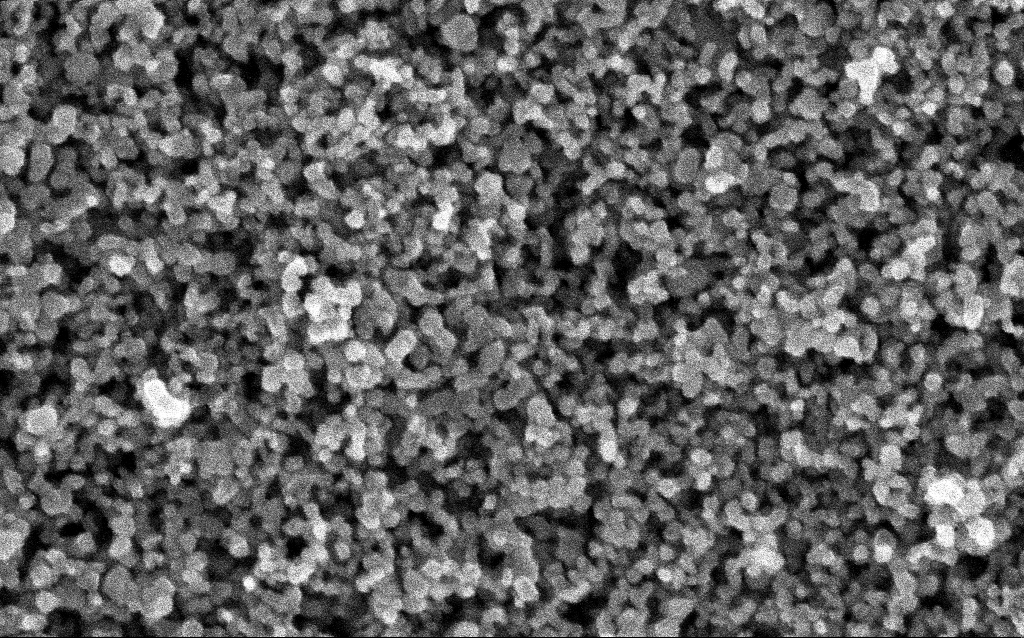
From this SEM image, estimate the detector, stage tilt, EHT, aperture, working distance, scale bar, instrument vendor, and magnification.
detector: InLens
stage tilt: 0°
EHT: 5 kV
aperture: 30 µm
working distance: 4.9 mm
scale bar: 100 nm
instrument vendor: Zeiss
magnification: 211.33 K X